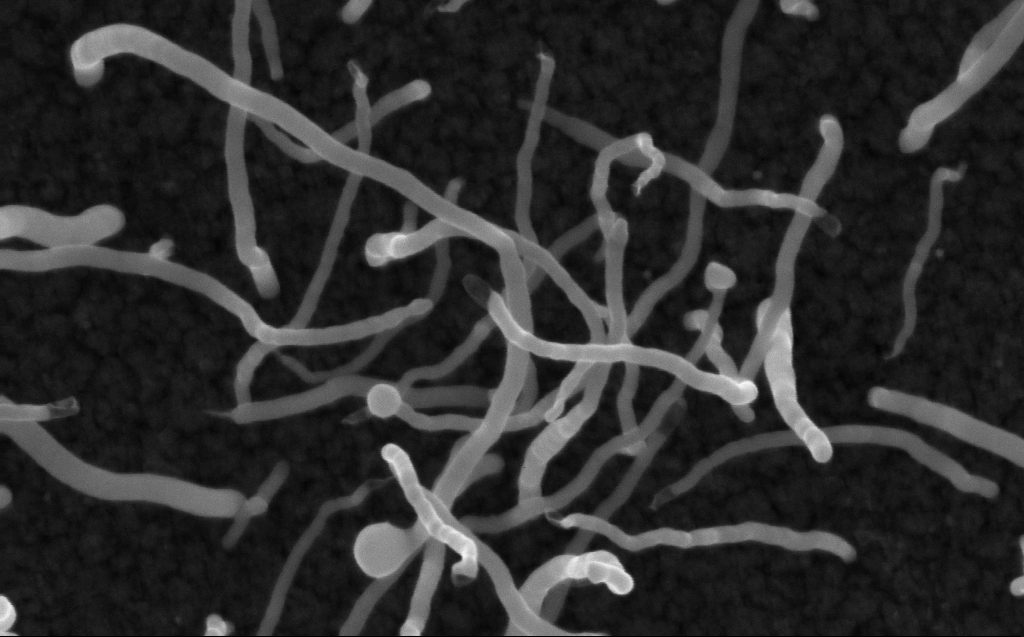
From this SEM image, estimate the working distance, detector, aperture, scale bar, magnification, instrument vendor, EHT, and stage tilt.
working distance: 3 mm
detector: InLens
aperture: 30 µm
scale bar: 100 nm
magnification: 155.83 K X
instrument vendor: Zeiss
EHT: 10 kV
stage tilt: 0°